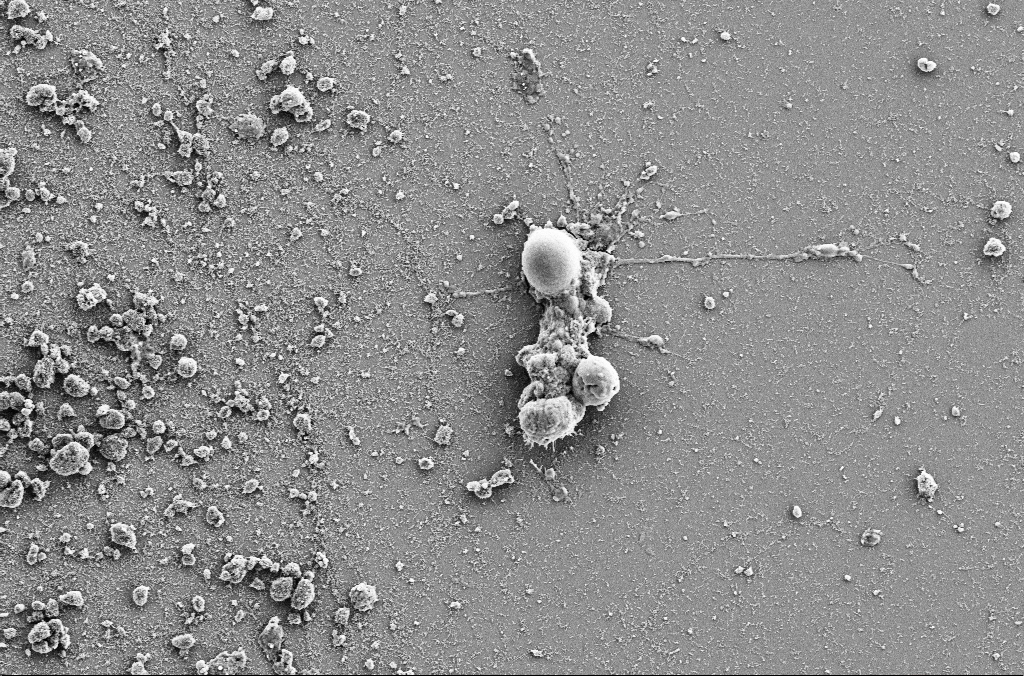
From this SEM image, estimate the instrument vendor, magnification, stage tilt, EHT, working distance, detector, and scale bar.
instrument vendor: Zeiss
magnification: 2.5 K X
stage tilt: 0°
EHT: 5 kV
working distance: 4 mm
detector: SE2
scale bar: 20000 nm